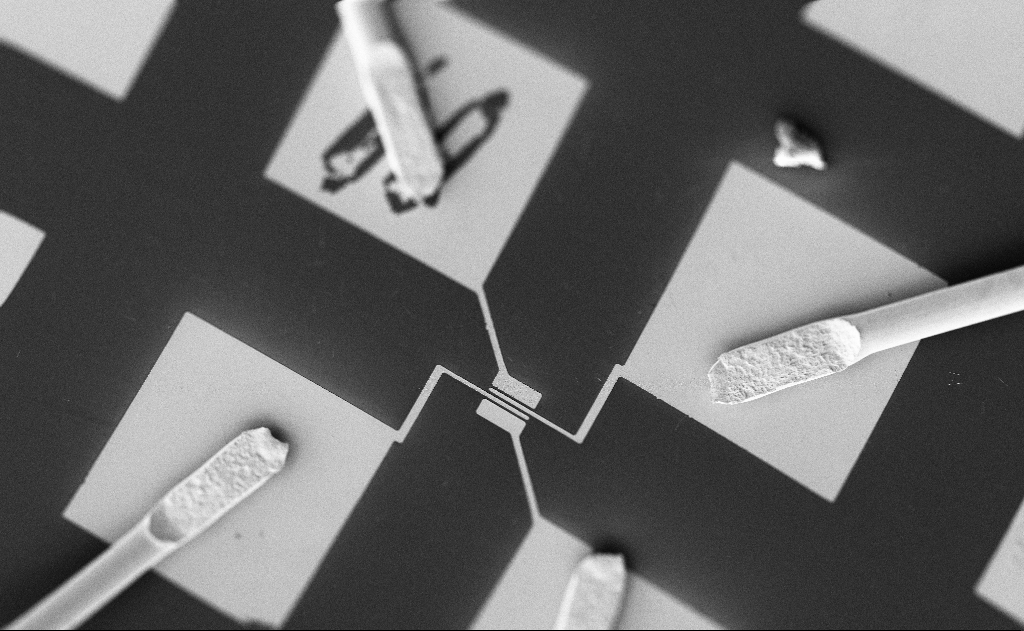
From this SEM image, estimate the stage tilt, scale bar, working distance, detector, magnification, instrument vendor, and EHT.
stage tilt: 0°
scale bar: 100000 nm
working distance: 21 mm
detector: SE2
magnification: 0.602 K X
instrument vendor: Zeiss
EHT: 5 kV